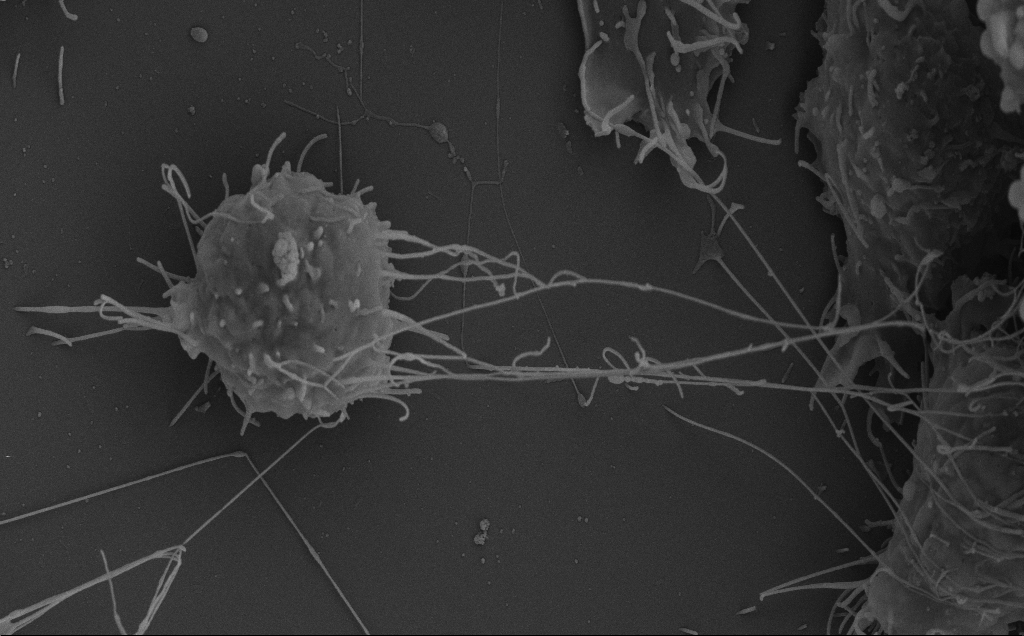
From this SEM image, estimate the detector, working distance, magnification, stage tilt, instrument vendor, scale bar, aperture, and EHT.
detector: SE2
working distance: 5 mm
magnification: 9.05 K X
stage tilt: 0.7°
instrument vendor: Zeiss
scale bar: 2000 nm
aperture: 30 µm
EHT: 10 kV